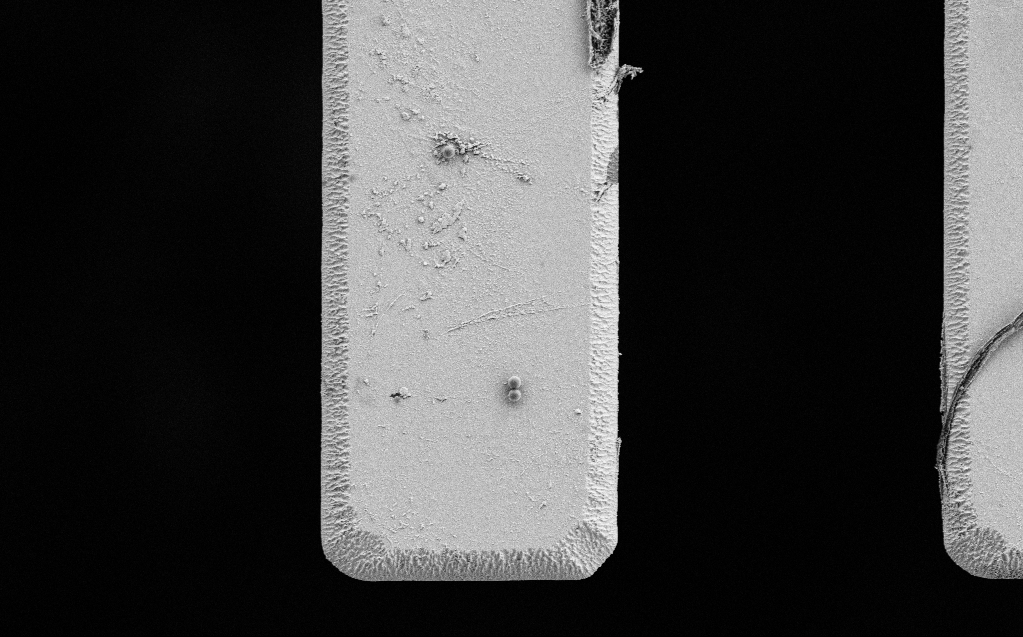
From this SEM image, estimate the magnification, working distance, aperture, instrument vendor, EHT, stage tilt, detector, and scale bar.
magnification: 5.72 K X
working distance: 7 mm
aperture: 30 µm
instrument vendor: Zeiss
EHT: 3 kV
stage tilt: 0°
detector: SE2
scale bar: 10000 nm